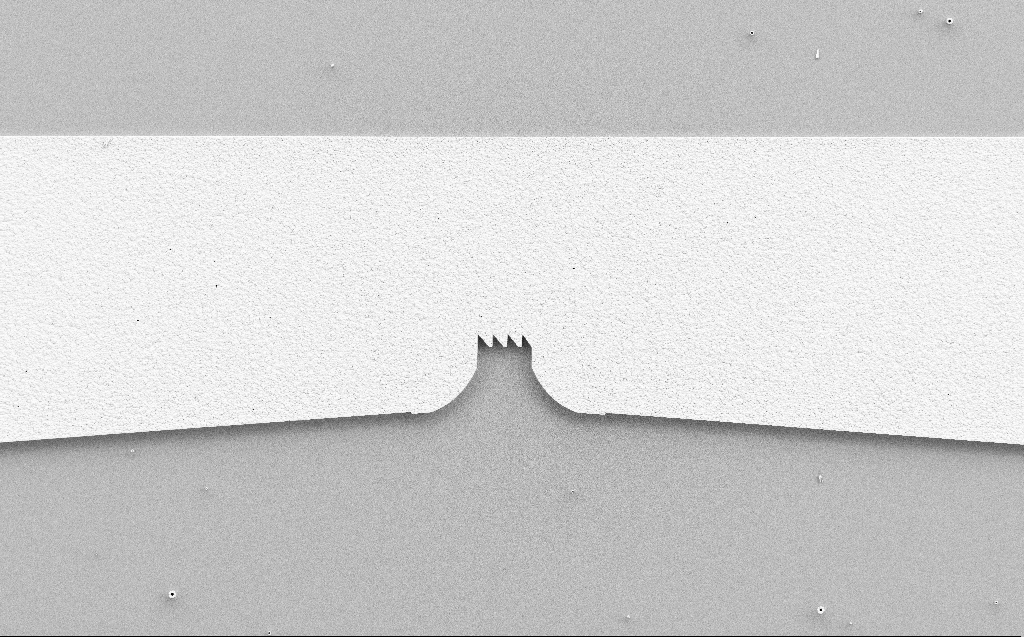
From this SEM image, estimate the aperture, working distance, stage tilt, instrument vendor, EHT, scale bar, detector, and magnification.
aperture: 30 µm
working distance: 6 mm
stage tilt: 0°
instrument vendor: Zeiss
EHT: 5 kV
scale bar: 100000 nm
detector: SE2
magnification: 0.479 K X